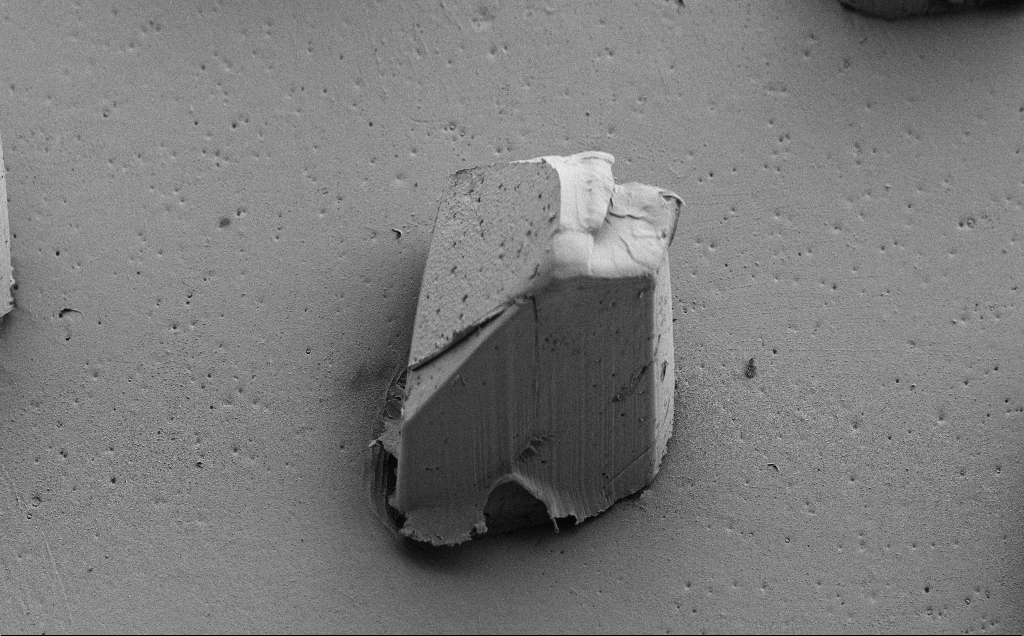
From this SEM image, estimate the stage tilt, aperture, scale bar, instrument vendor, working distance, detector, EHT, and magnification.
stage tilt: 39°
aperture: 30 µm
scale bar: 200000 nm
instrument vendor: Zeiss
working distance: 8 mm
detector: SE2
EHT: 5 kV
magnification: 0.265 K X